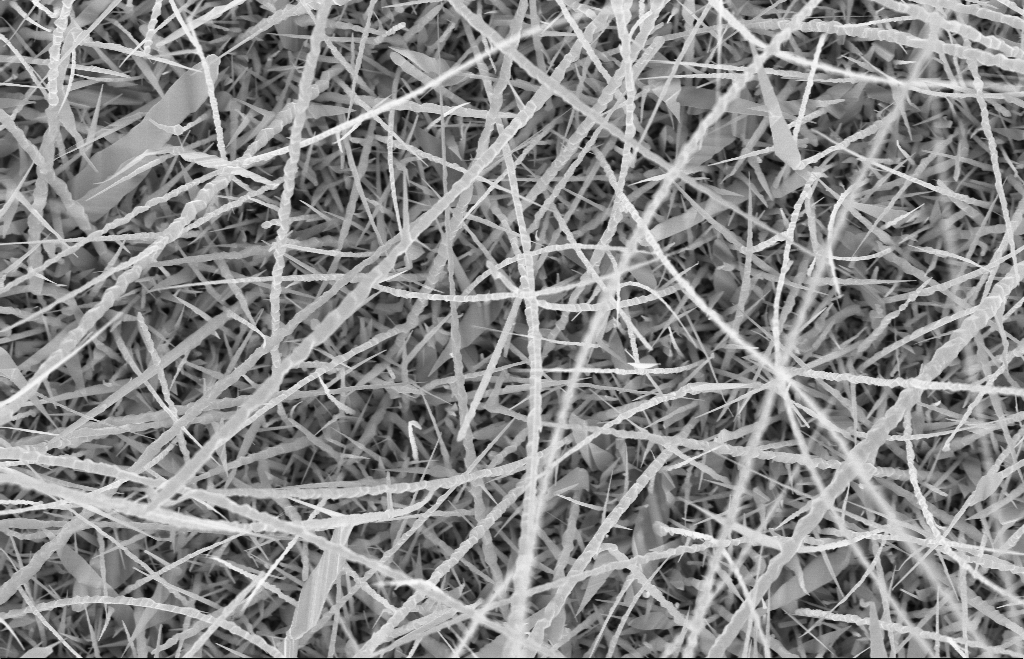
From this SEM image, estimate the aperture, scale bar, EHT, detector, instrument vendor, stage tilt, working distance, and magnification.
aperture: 30 µm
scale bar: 1000 nm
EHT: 10 kV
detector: InLens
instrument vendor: Zeiss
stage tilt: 0°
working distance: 9 mm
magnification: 20 K X